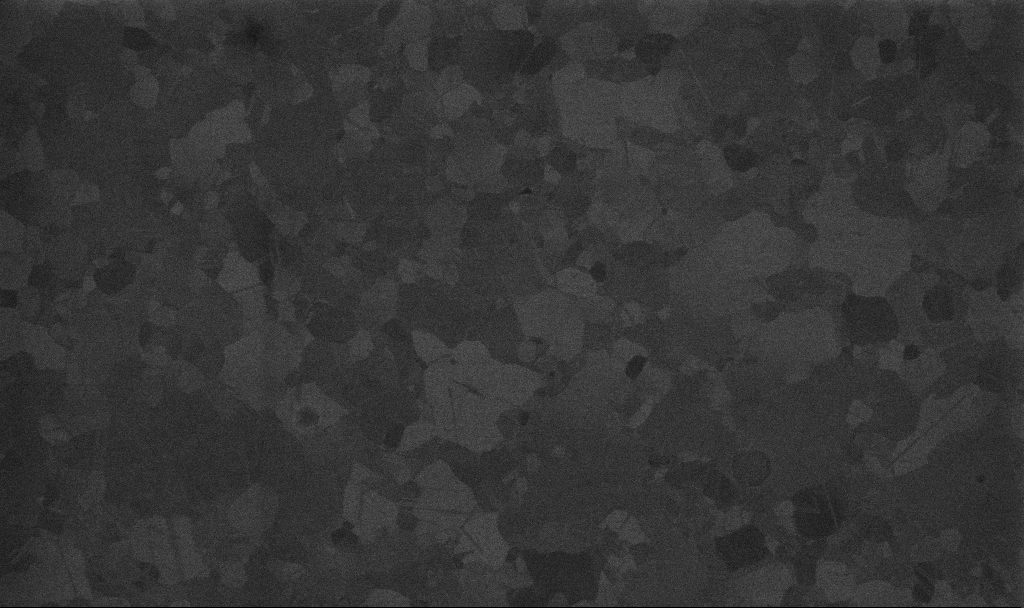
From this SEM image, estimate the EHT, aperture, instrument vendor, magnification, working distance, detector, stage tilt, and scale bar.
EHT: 10 kV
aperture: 30 µm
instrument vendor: Zeiss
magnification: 100 K X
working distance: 3.4 mm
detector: InLens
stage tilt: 0°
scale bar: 200 nm